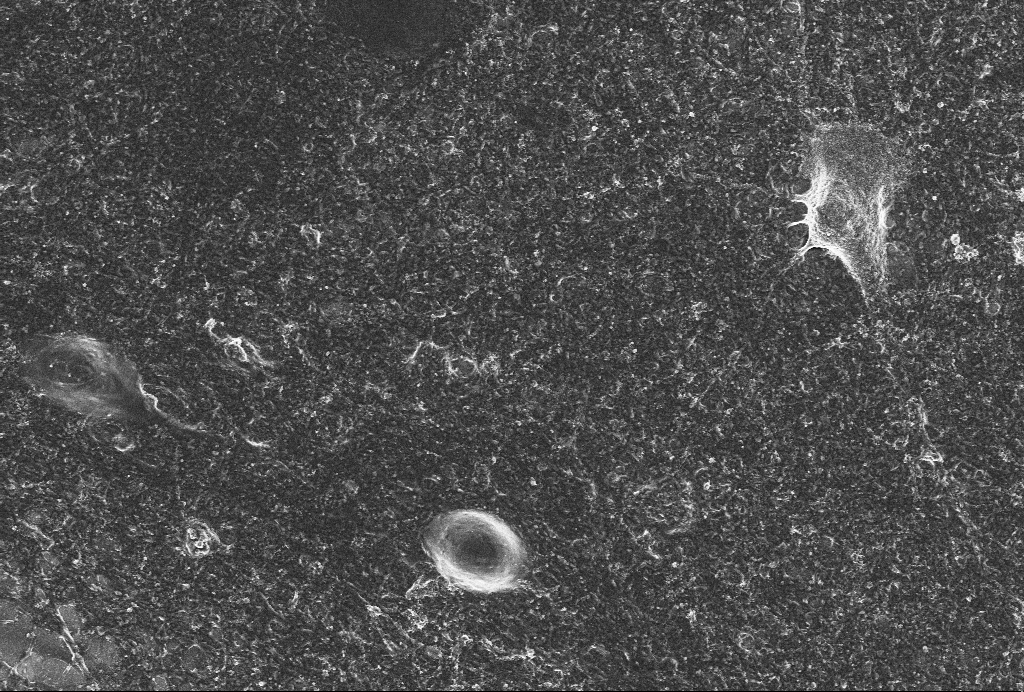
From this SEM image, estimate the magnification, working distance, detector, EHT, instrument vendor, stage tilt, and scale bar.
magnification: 3 K X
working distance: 6 mm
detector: InLens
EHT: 4 kV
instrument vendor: Zeiss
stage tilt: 0°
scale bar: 10000 nm